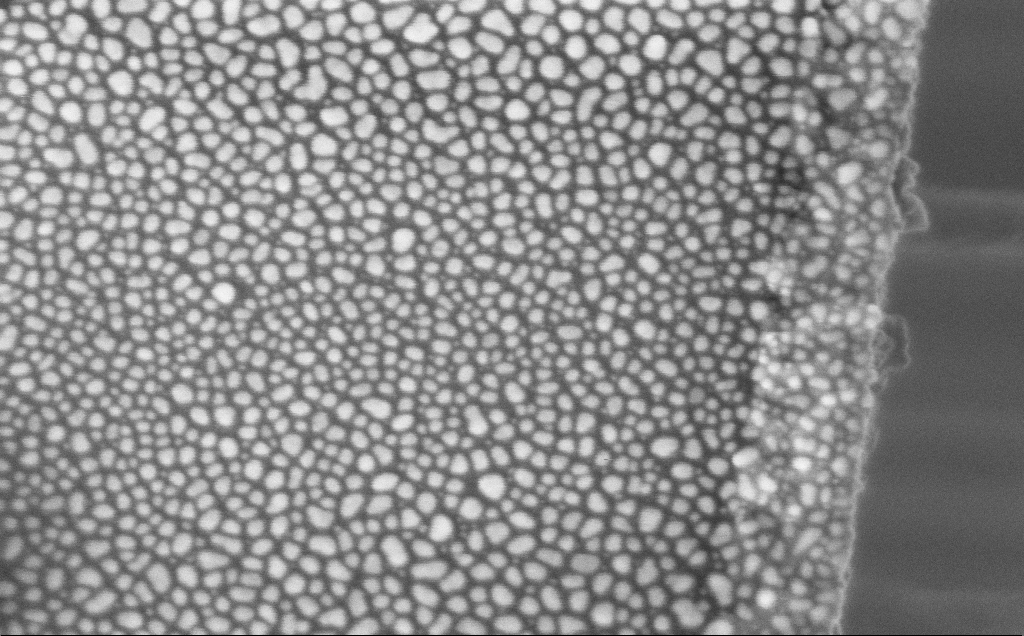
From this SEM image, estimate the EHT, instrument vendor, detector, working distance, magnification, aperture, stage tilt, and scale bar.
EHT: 5 kV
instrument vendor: Zeiss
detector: InLens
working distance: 12 mm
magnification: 183.52 K X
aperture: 30 µm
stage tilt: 0°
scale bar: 200 nm